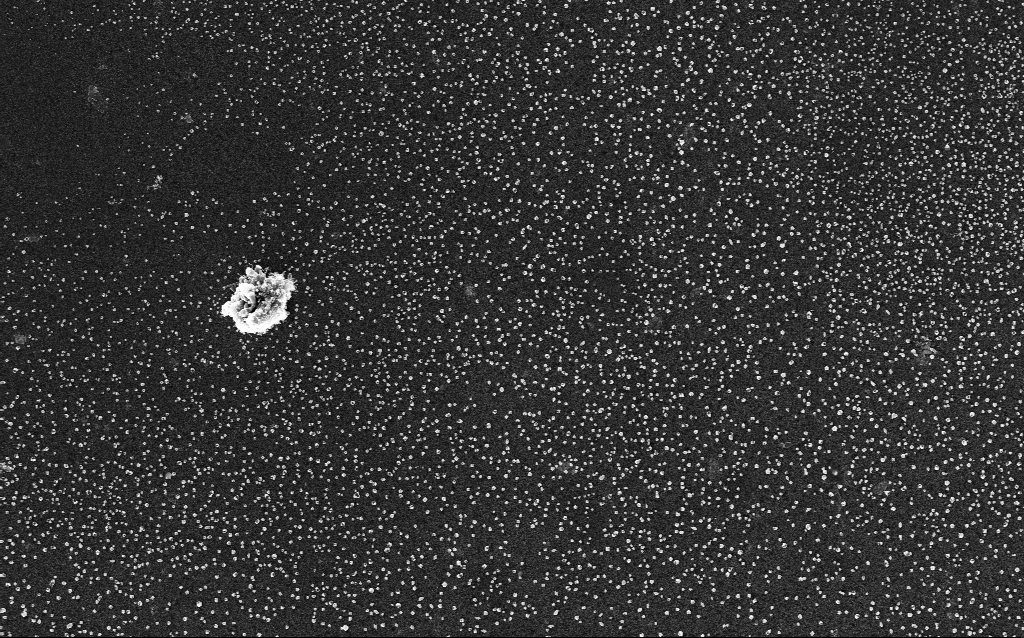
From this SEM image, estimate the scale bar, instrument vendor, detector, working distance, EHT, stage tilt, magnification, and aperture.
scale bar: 2000 nm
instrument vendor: Zeiss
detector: InLens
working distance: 2.8 mm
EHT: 5 kV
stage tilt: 0°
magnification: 10 K X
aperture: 30 µm